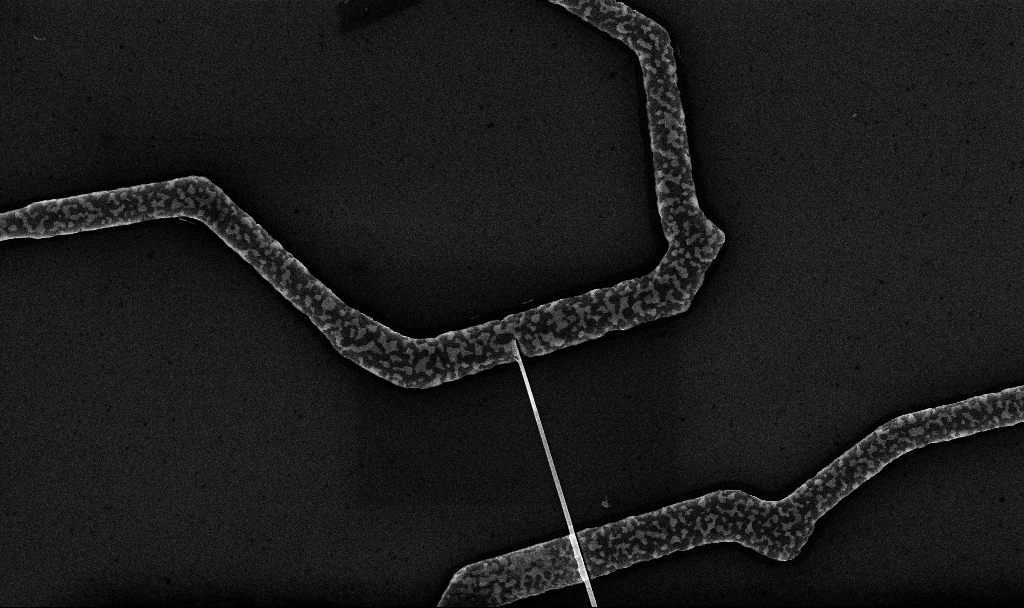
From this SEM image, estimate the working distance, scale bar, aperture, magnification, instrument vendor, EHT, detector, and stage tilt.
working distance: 6.7 mm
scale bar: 1000 nm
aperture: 30 µm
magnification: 20 K X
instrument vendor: Zeiss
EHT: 10 kV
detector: InLens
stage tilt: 0°